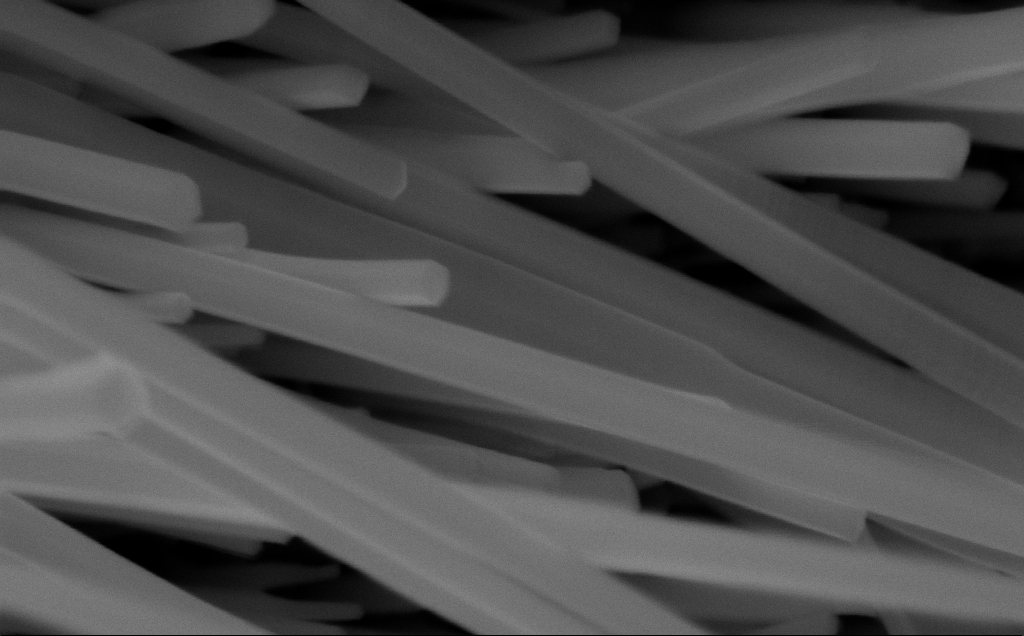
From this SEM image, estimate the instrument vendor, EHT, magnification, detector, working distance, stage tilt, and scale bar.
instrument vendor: Zeiss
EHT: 10 kV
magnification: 159.62 K X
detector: InLens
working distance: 6 mm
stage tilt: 0°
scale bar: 200 nm